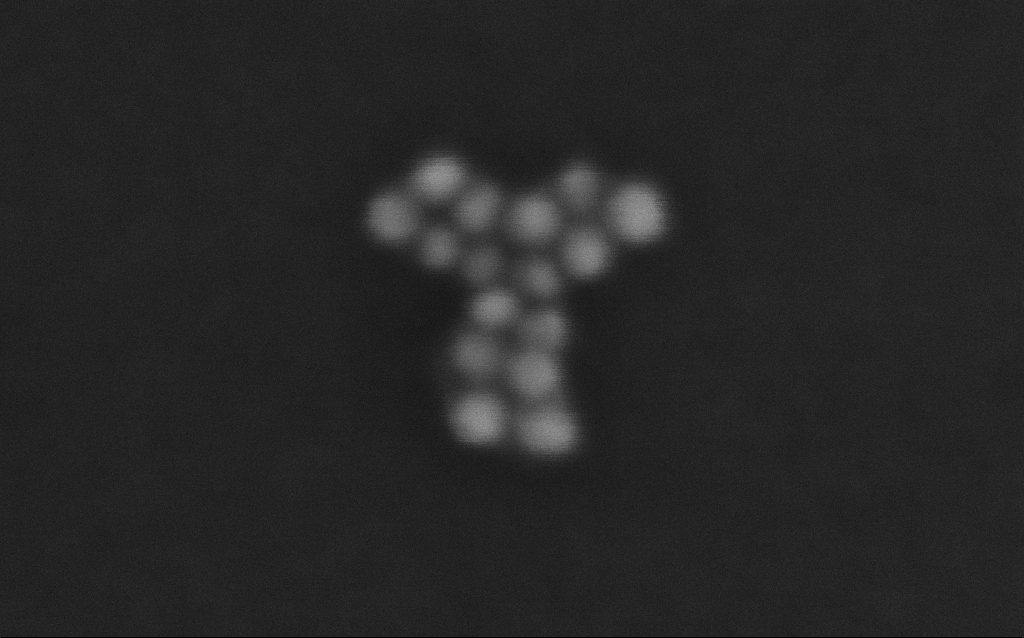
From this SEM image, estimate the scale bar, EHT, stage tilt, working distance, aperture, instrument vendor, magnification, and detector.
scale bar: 20 nm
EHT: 10 kV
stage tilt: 0°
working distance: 7 mm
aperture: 30 µm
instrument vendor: Zeiss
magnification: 1354.78 K X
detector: InLens